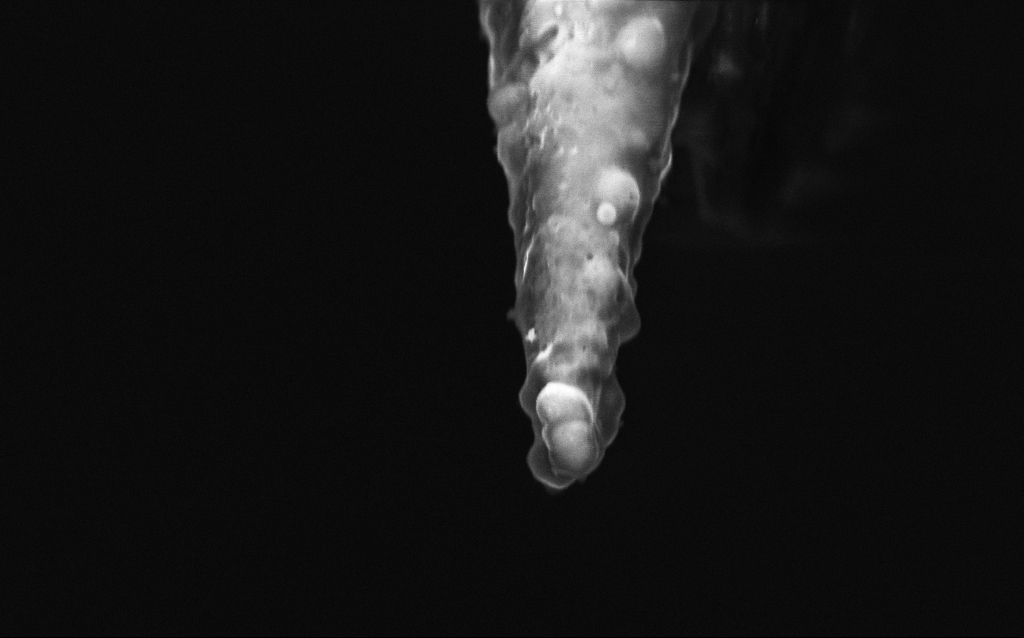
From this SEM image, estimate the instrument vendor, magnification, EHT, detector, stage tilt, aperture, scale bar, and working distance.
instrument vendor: Zeiss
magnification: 50 K X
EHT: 5 kV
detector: InLens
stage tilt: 43.9°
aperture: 30 µm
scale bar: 1000 nm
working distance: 6 mm